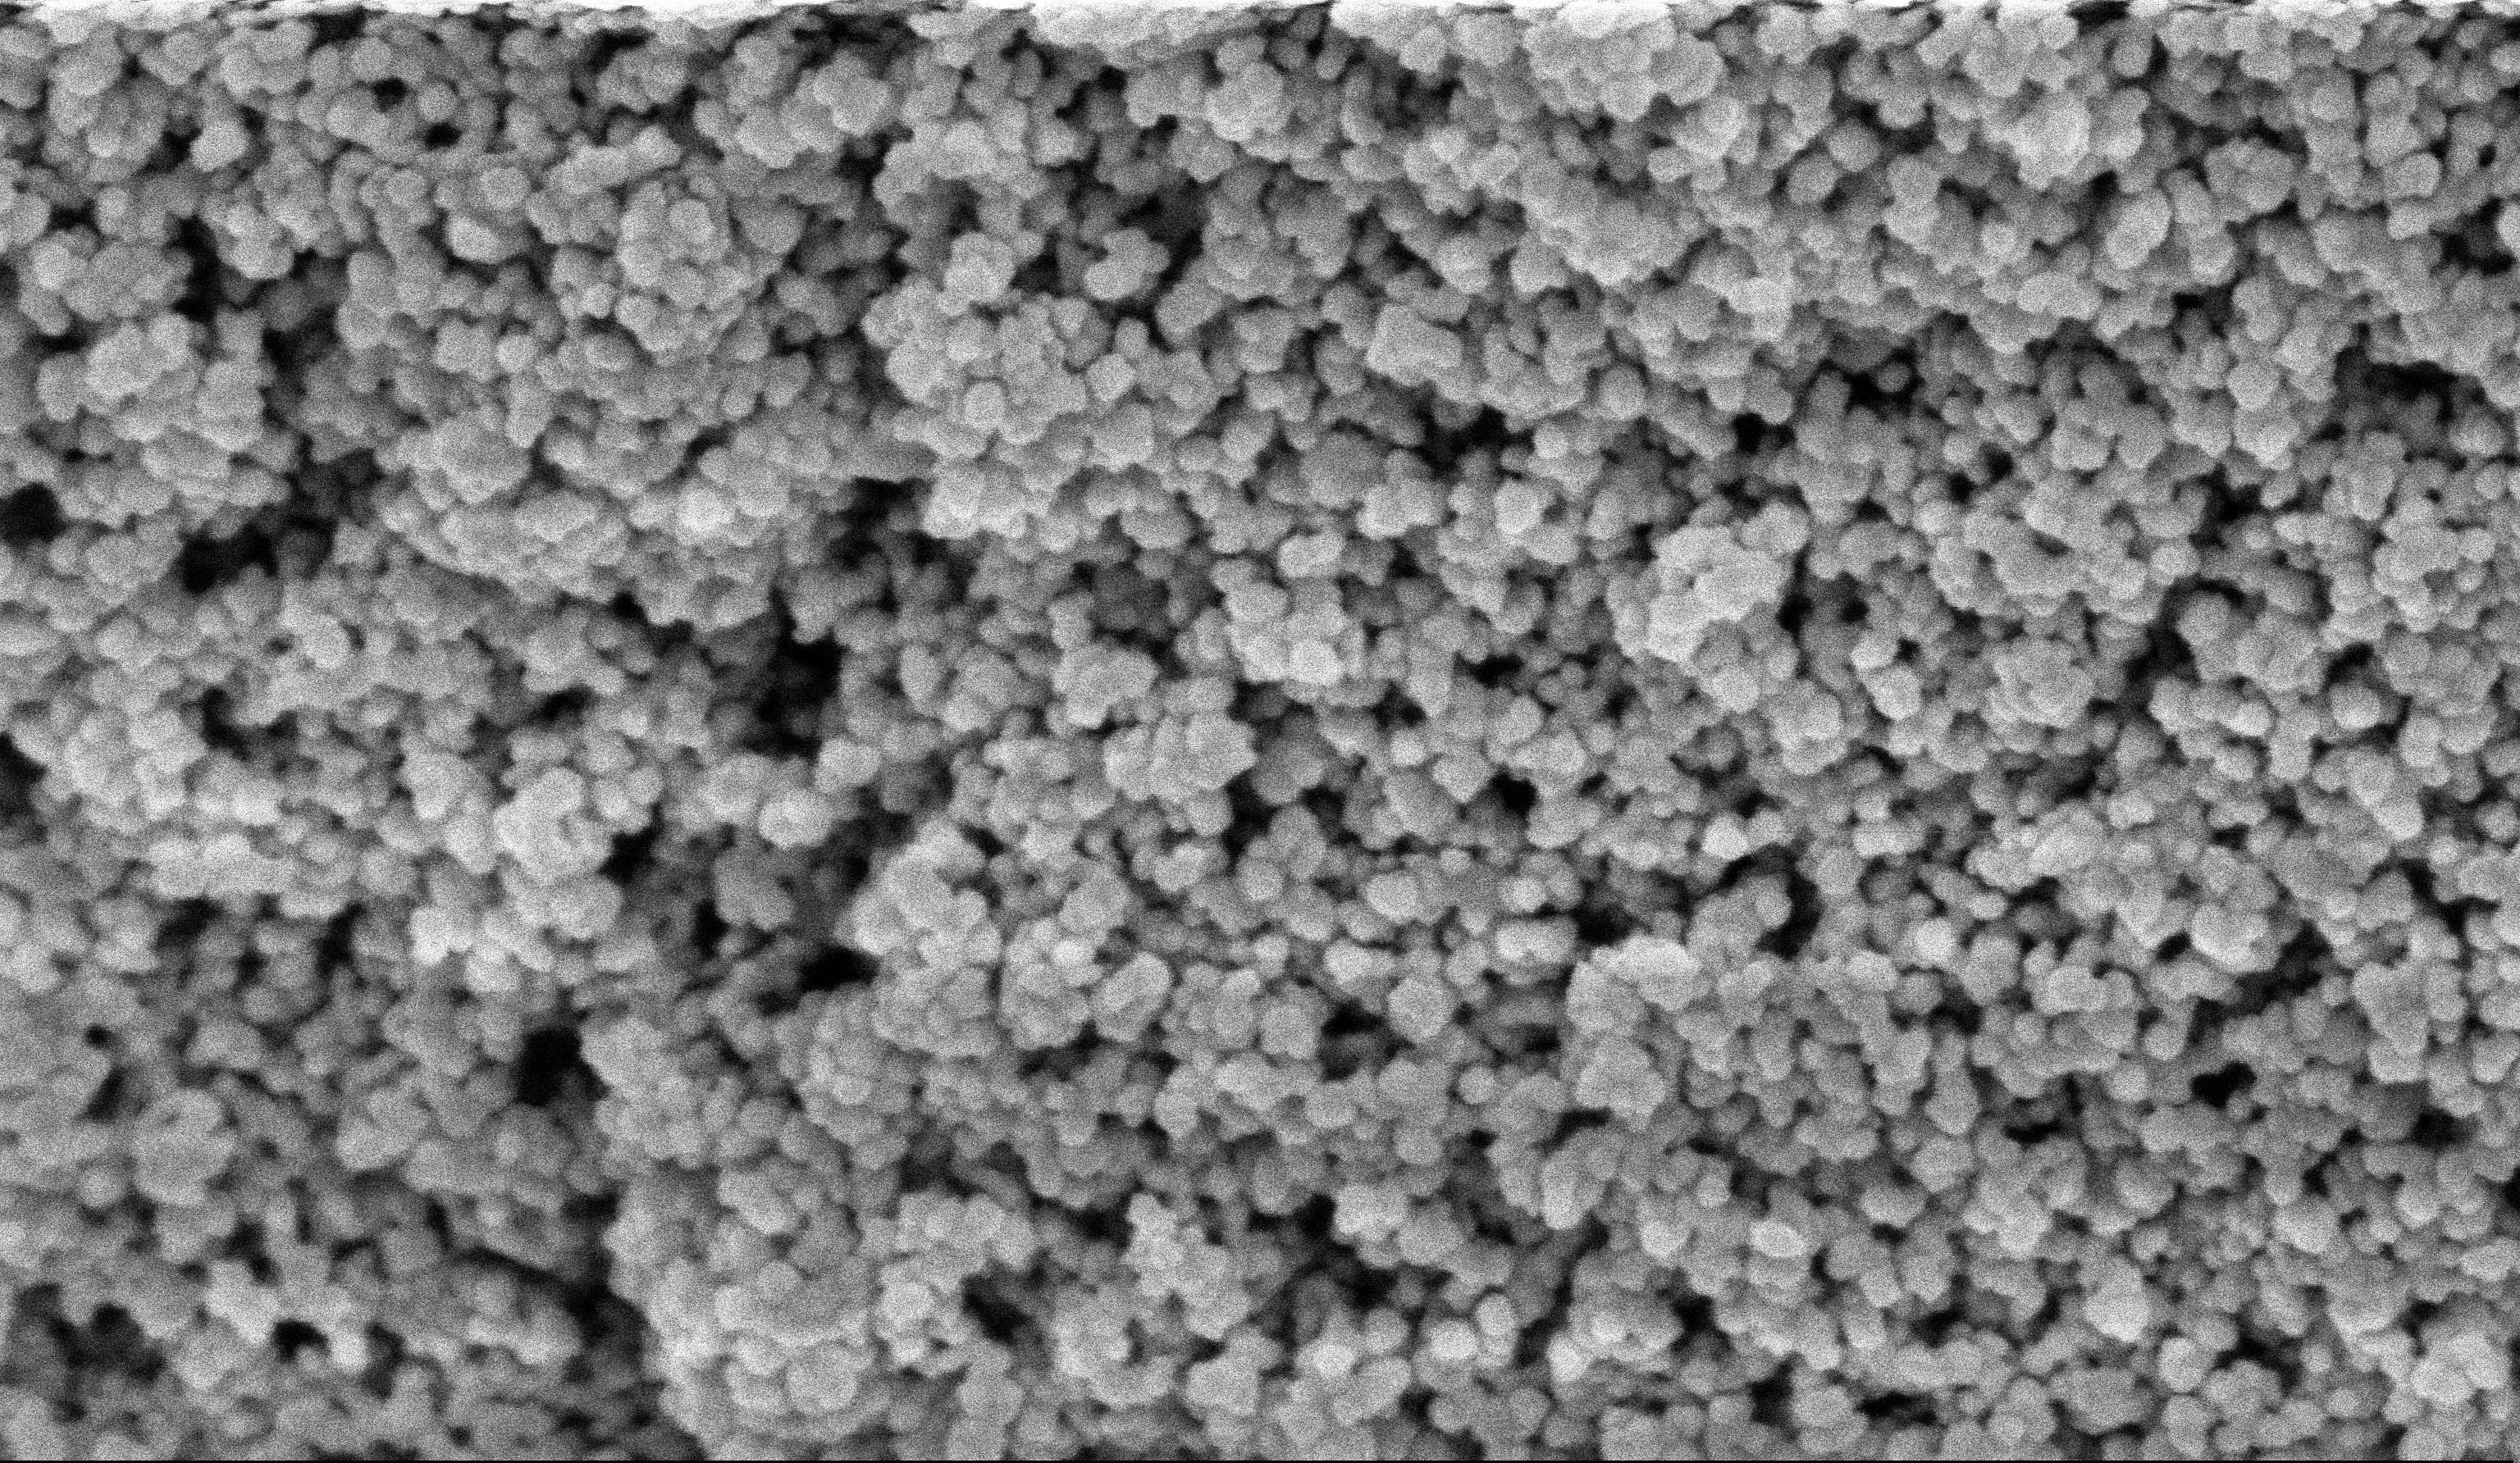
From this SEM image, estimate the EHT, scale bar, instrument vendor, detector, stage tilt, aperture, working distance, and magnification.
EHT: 5 kV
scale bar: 100 nm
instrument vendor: Zeiss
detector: InLens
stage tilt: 0°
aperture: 30 µm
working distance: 6 mm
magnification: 135 K X